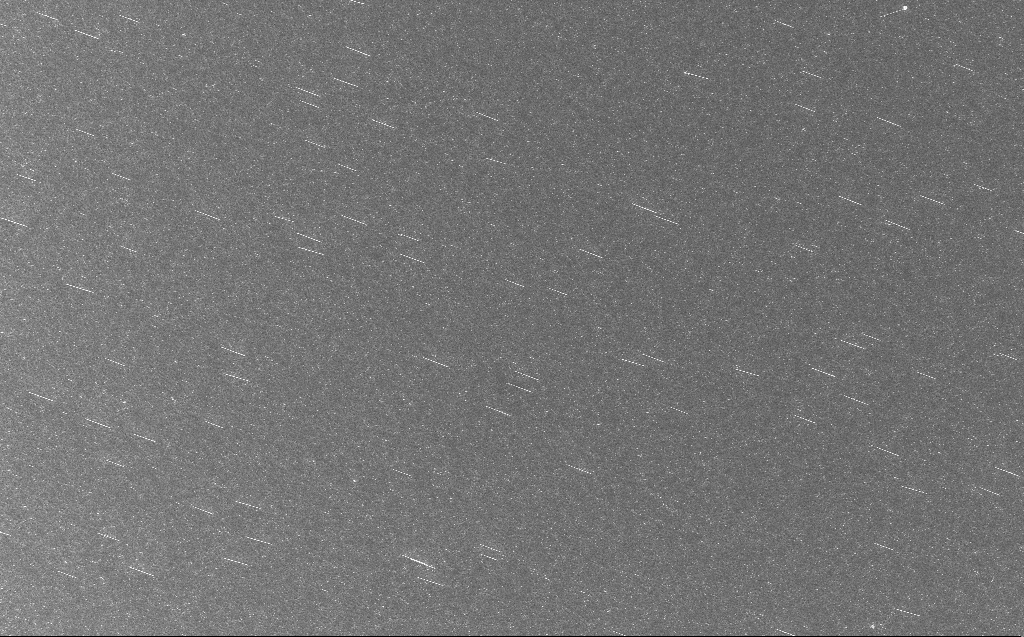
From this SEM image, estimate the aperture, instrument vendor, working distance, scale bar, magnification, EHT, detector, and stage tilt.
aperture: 30 µm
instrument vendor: Zeiss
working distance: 3 mm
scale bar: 10000 nm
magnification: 2 K X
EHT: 10 kV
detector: InLens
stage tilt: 0°